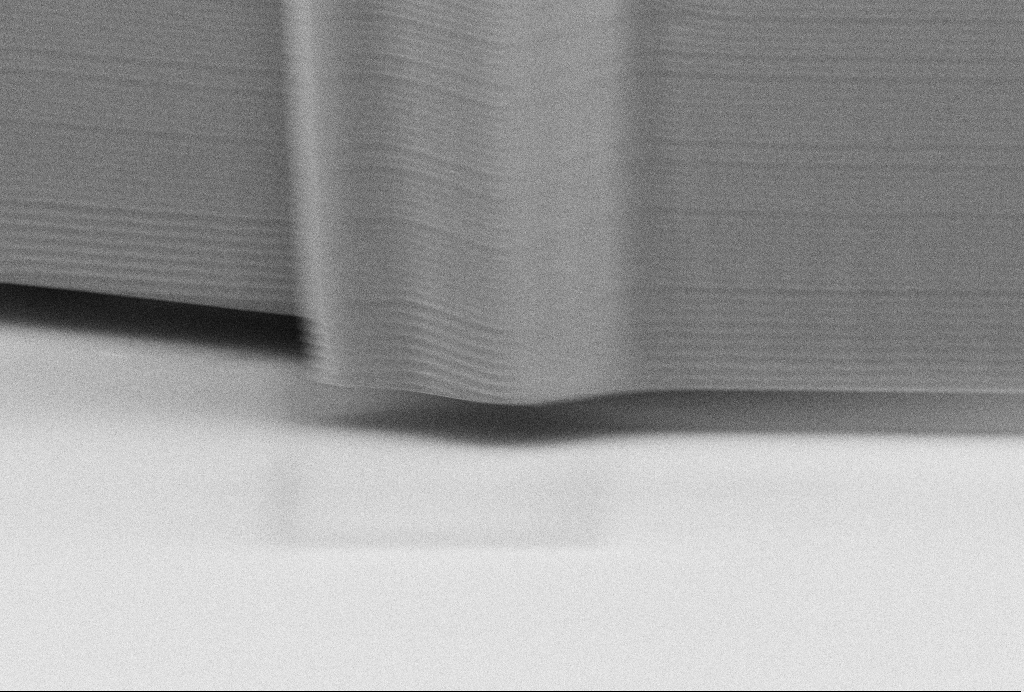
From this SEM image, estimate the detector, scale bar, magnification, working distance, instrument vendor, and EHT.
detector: SE2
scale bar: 2000 nm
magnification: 15.69 K X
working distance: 14.1 mm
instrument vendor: Zeiss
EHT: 10 kV